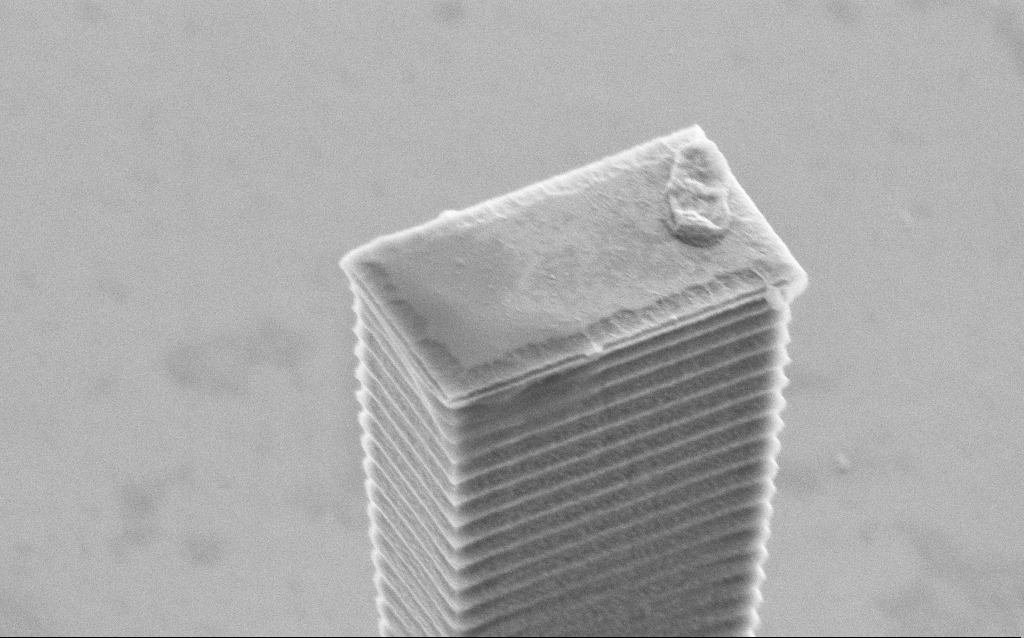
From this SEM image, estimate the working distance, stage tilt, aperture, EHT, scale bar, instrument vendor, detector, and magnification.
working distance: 12 mm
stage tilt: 45°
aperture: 30 µm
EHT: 5 kV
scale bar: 1000 nm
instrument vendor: Zeiss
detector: SE2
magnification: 30.26 K X